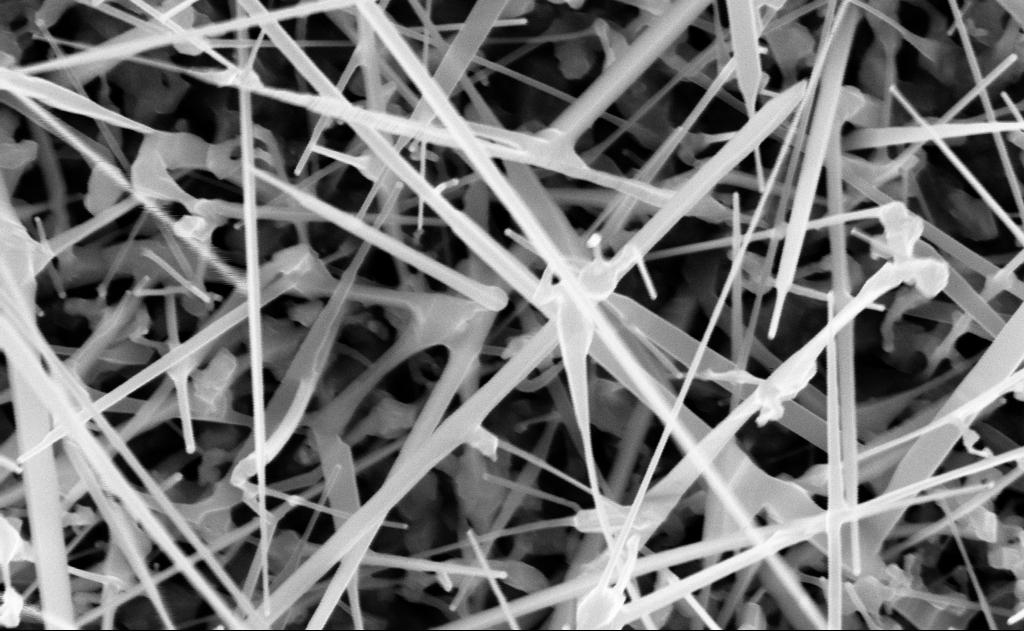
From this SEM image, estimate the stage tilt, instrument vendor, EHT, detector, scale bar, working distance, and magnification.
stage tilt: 0°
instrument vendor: Zeiss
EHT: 10 kV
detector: InLens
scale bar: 200 nm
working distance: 11 mm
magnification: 80 K X